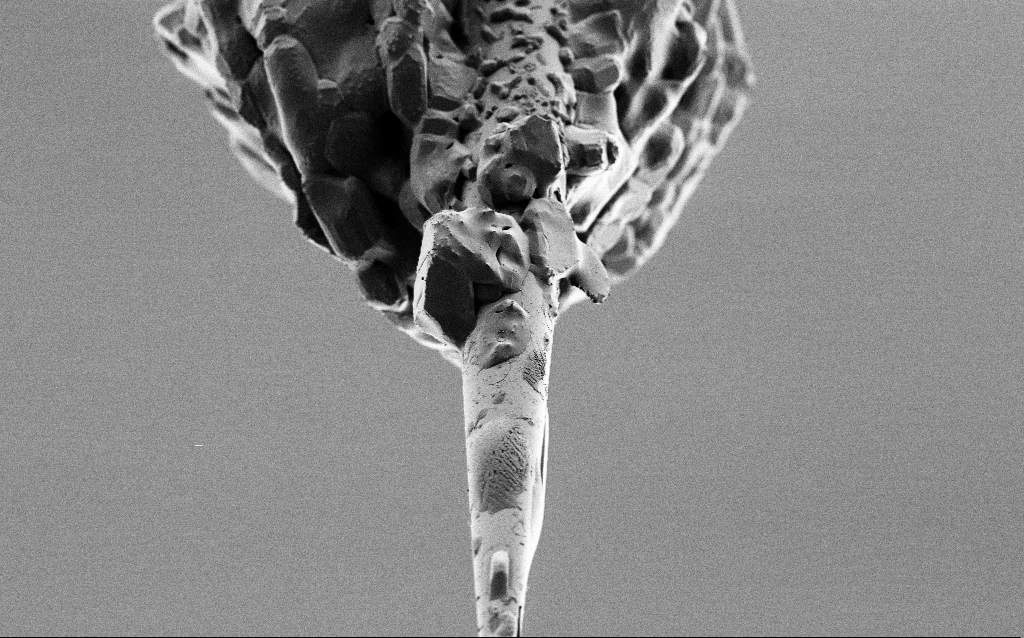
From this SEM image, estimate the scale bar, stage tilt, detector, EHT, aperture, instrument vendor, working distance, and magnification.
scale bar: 10000 nm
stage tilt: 45°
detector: SE2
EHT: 1 kV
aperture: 30 µm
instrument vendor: Zeiss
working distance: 6.5 mm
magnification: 2.5 K X